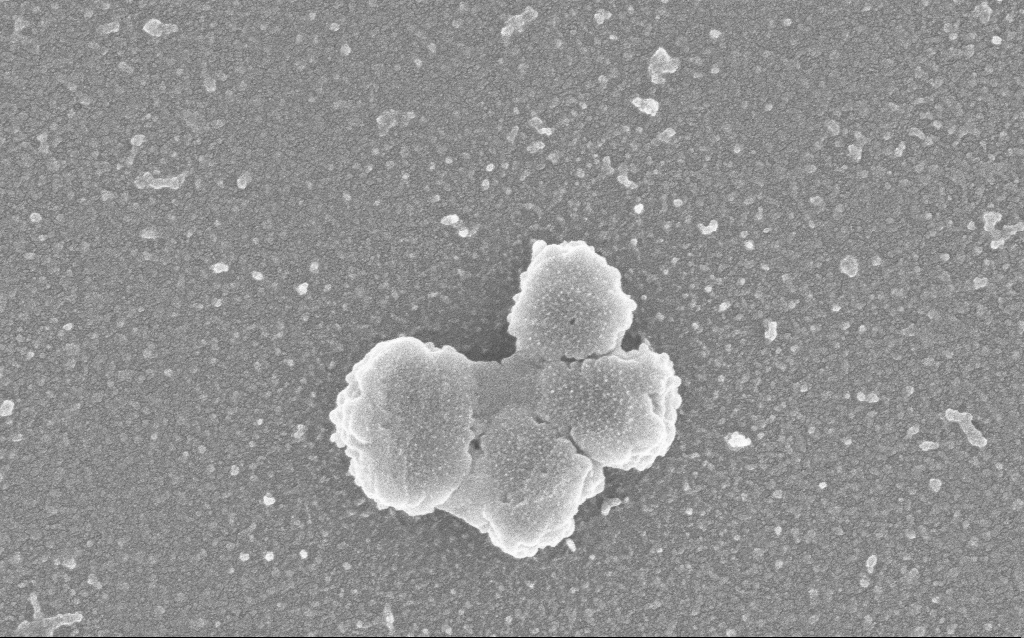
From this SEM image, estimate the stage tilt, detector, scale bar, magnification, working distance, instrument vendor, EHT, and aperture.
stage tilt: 0°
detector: InLens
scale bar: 200 nm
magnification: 100 K X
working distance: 2.5 mm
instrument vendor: Zeiss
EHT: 3 kV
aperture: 30 µm